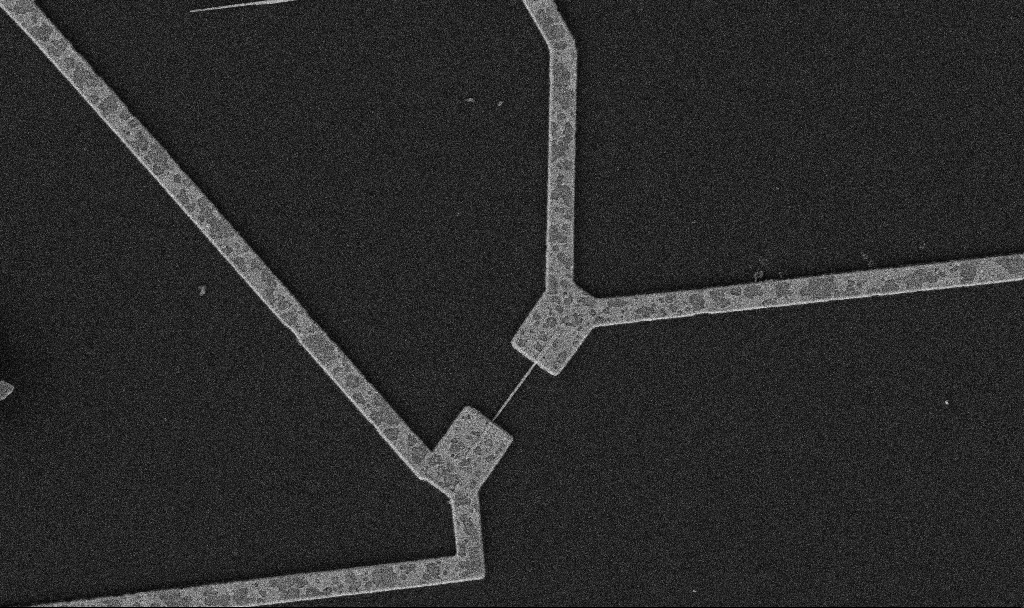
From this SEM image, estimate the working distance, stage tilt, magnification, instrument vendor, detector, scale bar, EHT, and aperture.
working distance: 10.7 mm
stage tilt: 0°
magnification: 10 K X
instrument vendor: Zeiss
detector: SE2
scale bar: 2000 nm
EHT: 5 kV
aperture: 30 µm